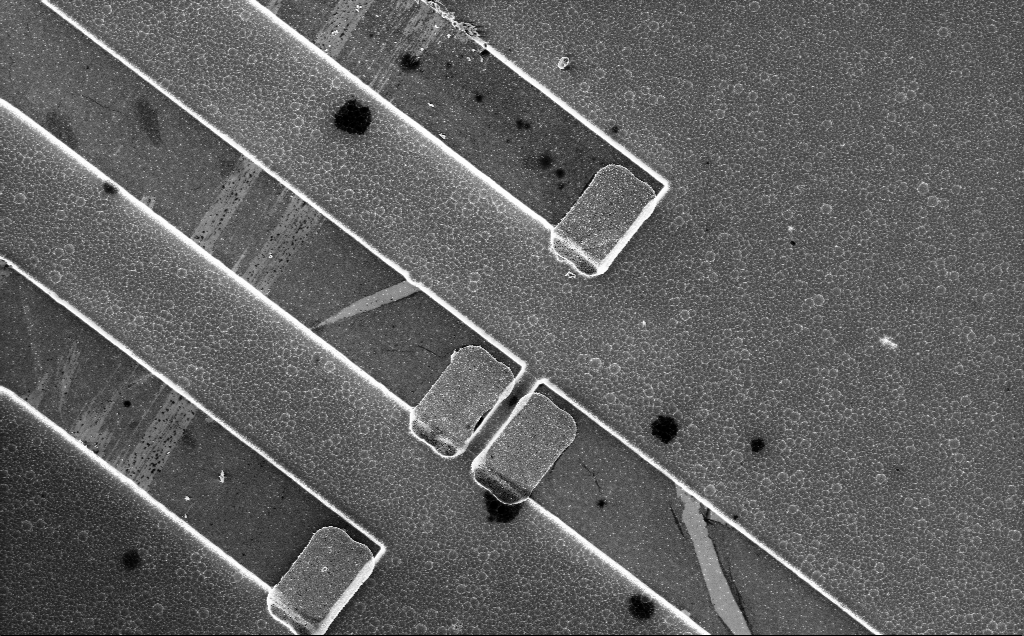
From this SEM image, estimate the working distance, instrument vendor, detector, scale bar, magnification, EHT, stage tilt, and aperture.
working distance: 10 mm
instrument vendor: Zeiss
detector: InLens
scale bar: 10000 nm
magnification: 2.12 K X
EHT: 5 kV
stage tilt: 0°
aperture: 30 µm